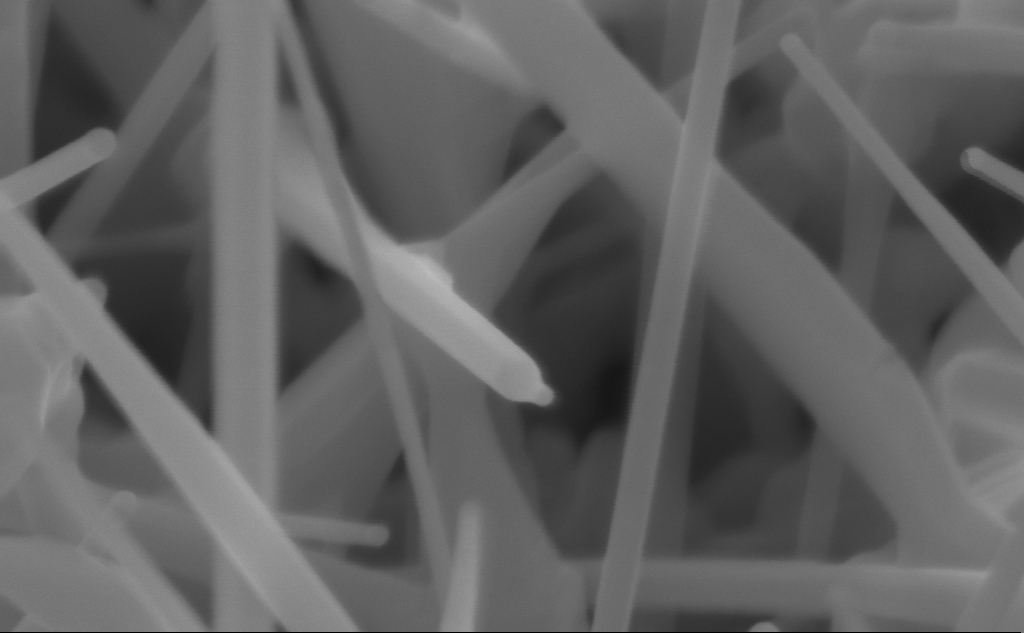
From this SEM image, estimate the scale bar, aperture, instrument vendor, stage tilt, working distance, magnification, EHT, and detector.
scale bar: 200 nm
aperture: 30 µm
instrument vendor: Zeiss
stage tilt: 45°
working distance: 4 mm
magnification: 296.9 K X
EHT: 10 kV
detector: InLens